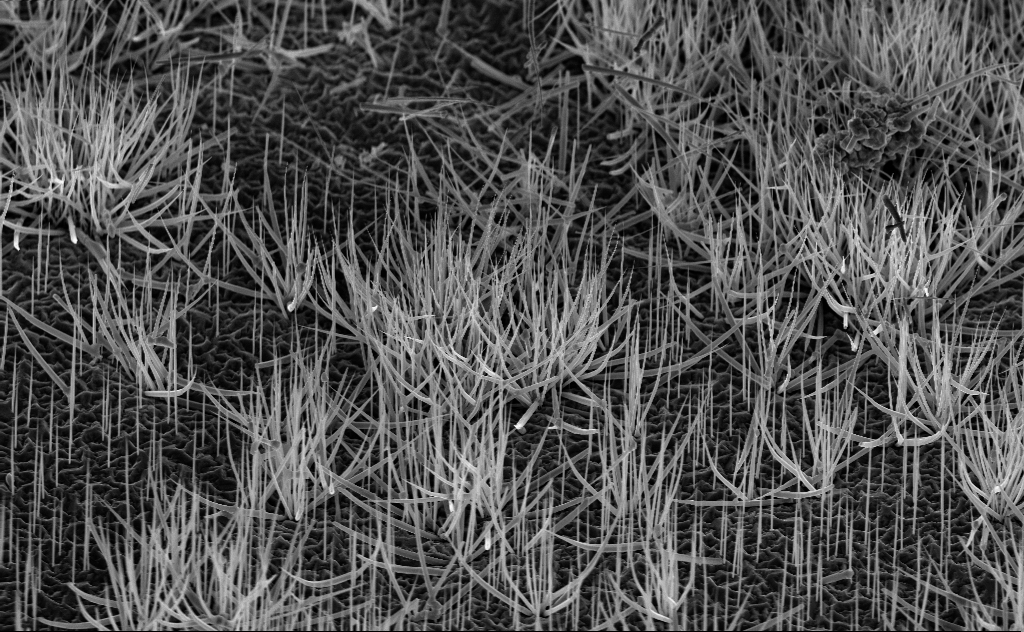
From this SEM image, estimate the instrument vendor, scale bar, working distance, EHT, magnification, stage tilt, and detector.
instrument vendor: Zeiss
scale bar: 10000 nm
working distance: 7 mm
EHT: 10 kV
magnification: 4.79 K X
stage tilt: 45°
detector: InLens